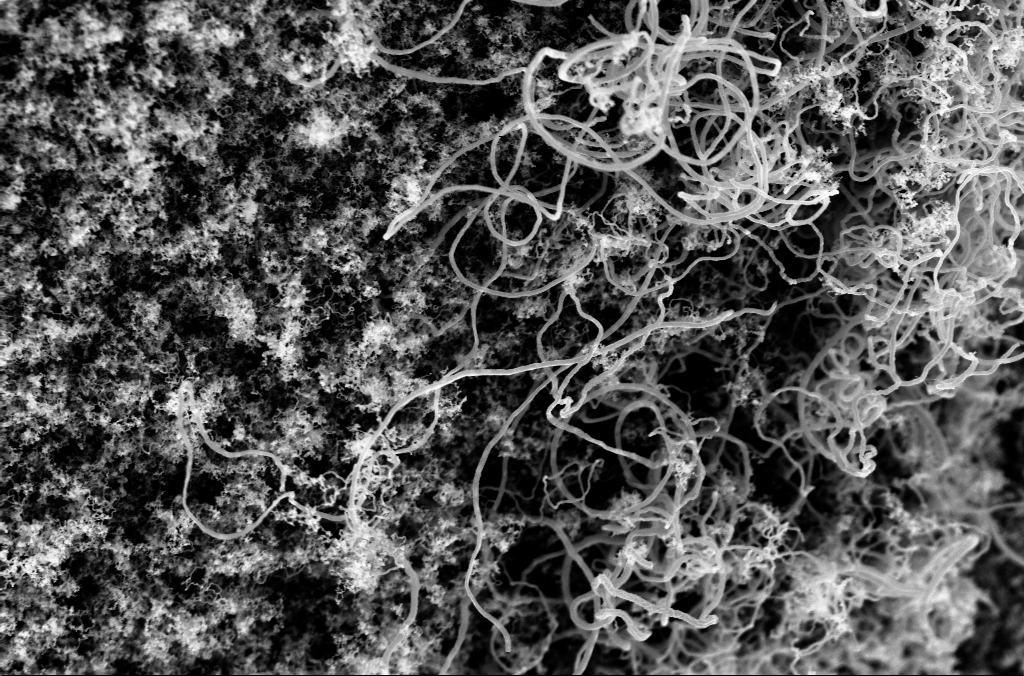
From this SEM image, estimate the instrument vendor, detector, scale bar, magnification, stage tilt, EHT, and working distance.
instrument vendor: Zeiss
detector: InLens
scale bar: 1000 nm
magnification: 25 K X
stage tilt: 0°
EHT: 3 kV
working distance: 5.1 mm